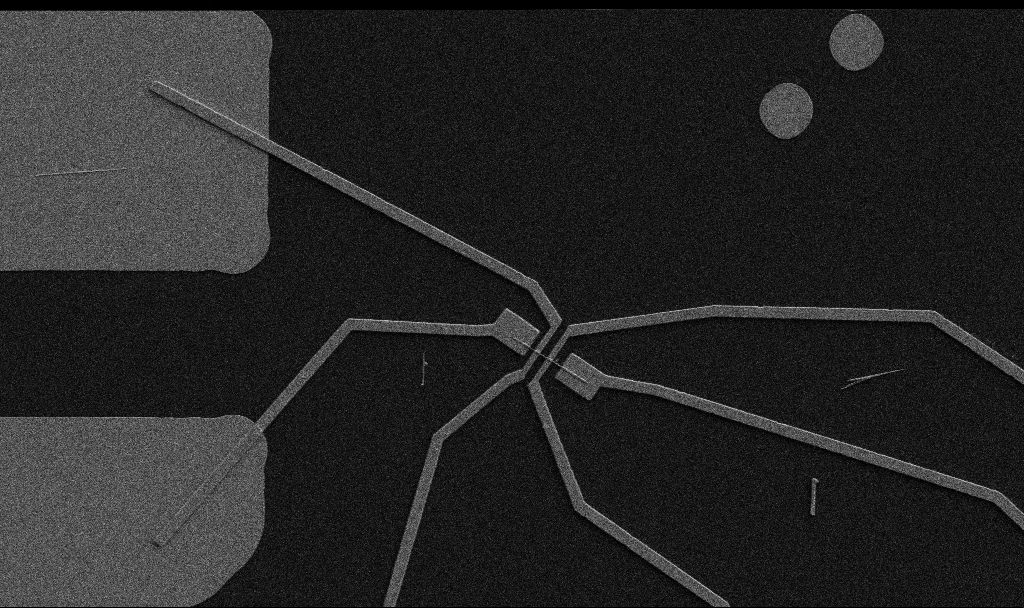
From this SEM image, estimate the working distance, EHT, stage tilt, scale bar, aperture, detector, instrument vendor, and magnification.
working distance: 10.7 mm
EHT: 5 kV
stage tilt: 0°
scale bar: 10000 nm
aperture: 30 µm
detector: SE2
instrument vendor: Zeiss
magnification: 5 K X